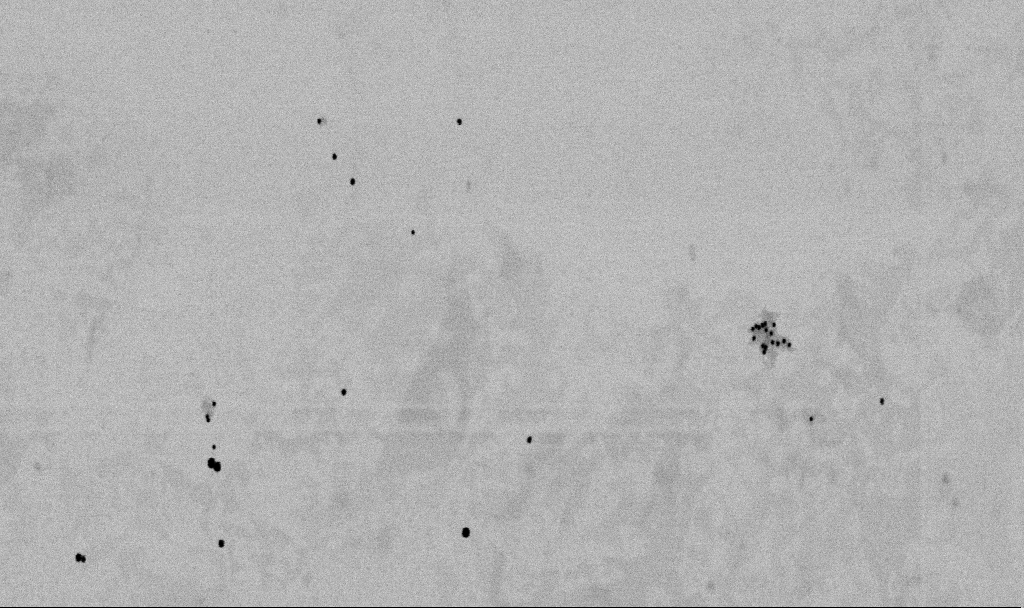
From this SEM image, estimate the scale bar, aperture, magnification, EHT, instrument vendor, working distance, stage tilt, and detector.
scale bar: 1000 nm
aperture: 30 µm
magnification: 50 K X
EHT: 2 kV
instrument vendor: Zeiss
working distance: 6.5 mm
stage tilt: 0°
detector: SE2